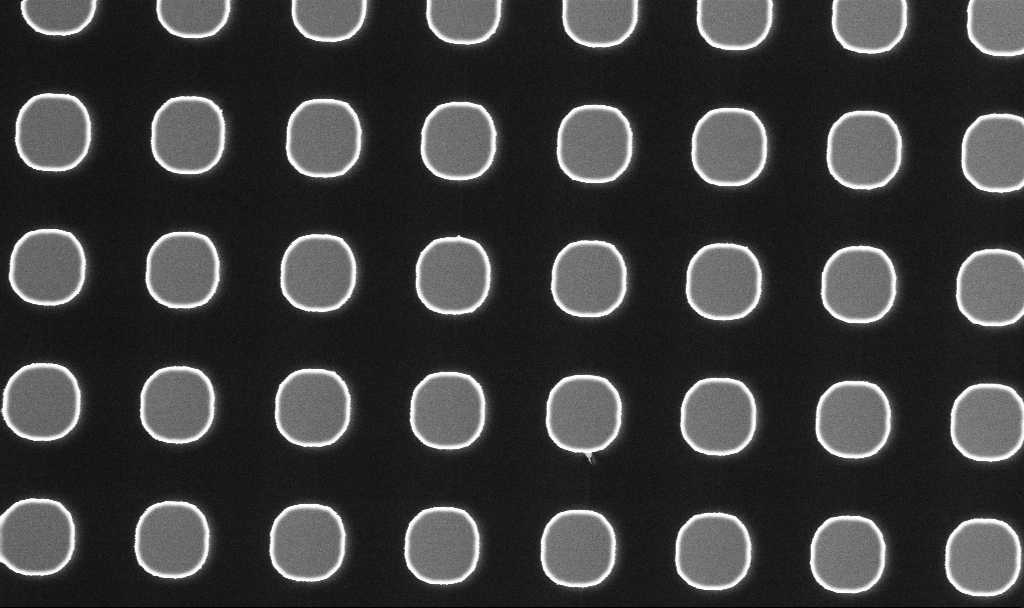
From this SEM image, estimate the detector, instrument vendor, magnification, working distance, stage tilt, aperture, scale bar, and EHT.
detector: InLens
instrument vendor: Zeiss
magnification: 12.52 K X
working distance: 2.9 mm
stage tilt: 0°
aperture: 30 µm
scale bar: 1000 nm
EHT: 3 kV